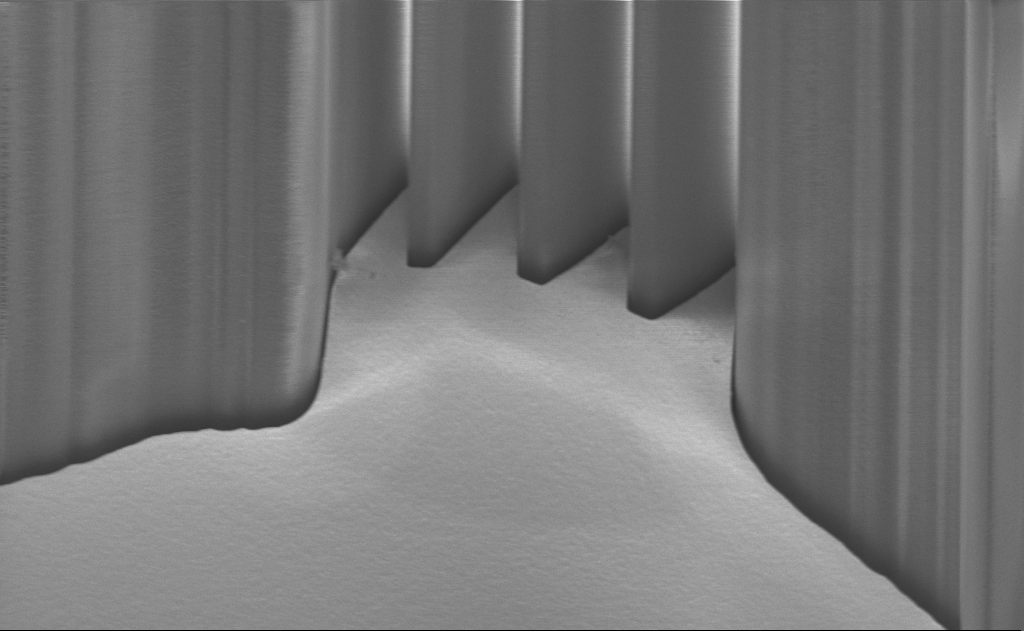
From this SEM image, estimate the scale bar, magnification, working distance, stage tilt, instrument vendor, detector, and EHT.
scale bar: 20000 nm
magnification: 3.51 K X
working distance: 8 mm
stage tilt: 45°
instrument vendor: Zeiss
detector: InLens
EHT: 1.5 kV